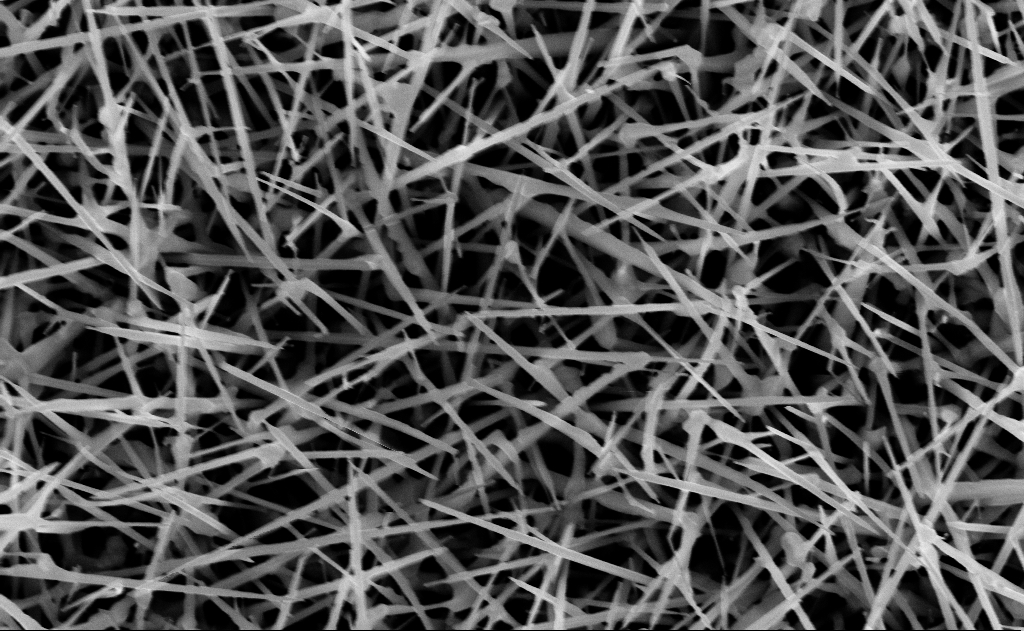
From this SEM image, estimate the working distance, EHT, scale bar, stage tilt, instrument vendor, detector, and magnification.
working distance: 13 mm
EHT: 10 kV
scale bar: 1000 nm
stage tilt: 0°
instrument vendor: Zeiss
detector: InLens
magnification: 40 K X